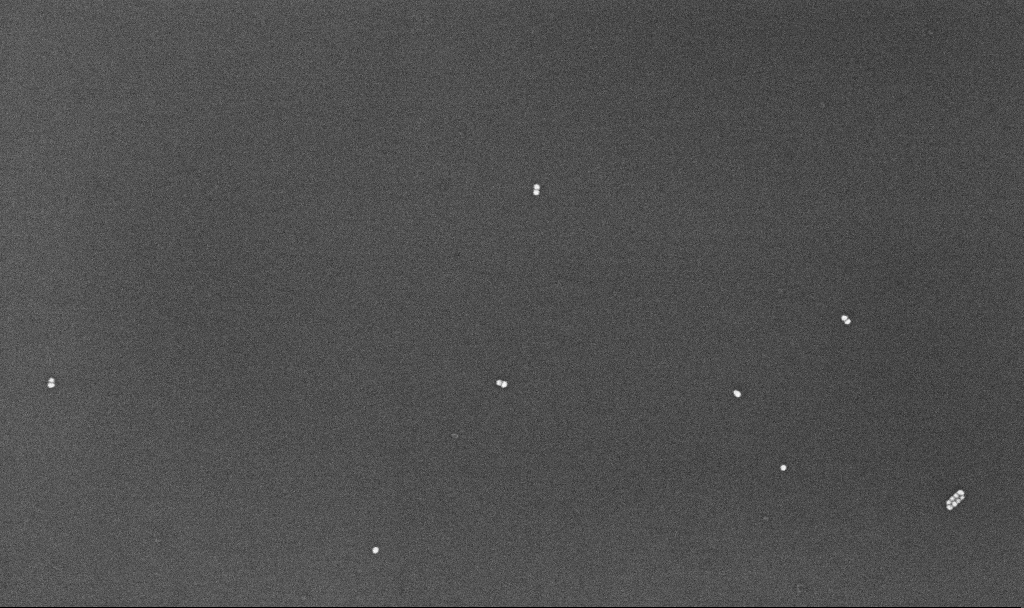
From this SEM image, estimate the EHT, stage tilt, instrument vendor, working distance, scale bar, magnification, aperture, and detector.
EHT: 10 kV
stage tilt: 0°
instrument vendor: Zeiss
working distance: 4.3 mm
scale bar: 200 nm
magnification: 118.51 K X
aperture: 30 µm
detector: InLens